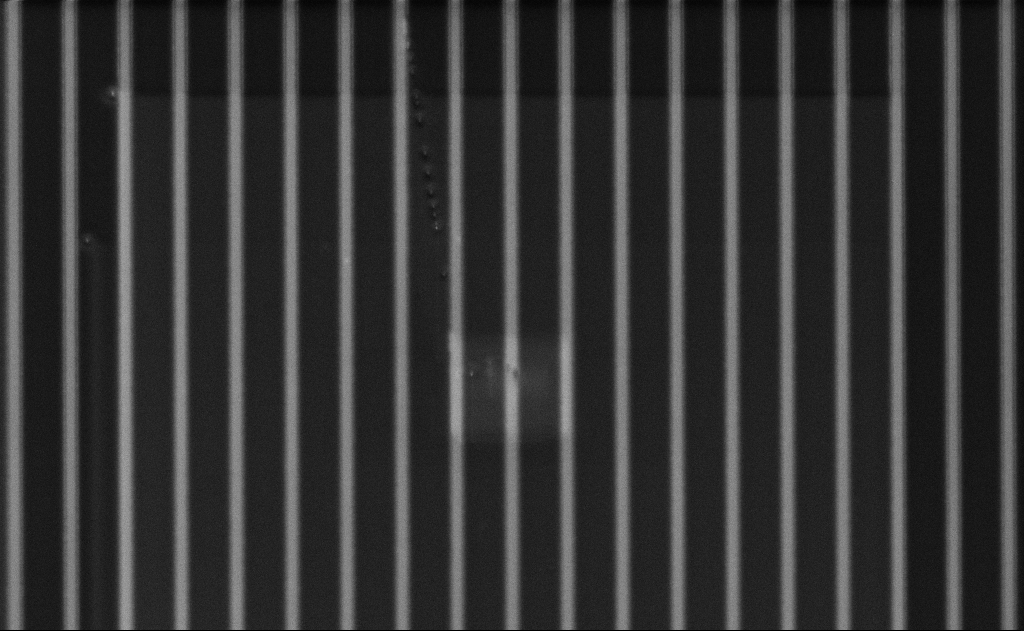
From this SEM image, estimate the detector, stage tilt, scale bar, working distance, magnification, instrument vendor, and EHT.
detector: SE2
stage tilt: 50°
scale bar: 10000 nm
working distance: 18 mm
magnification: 5.01 K X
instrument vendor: Zeiss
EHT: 5 kV